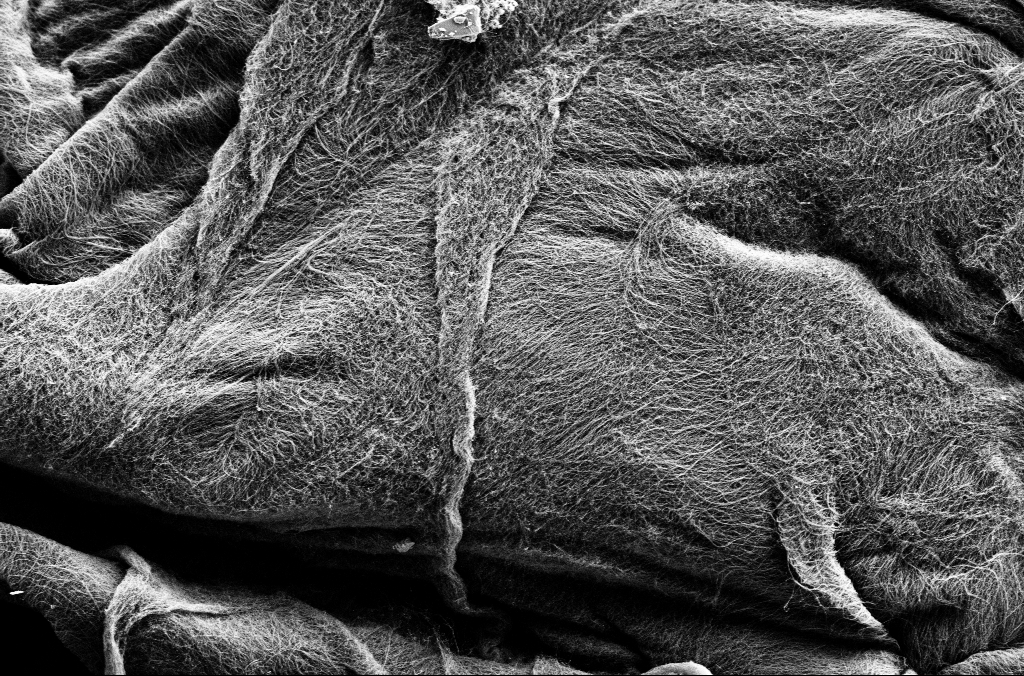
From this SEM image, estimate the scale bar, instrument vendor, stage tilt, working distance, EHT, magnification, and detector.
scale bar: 2000 nm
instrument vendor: Zeiss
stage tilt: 0°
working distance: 5.4 mm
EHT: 1.8 kV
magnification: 10 K X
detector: SE2